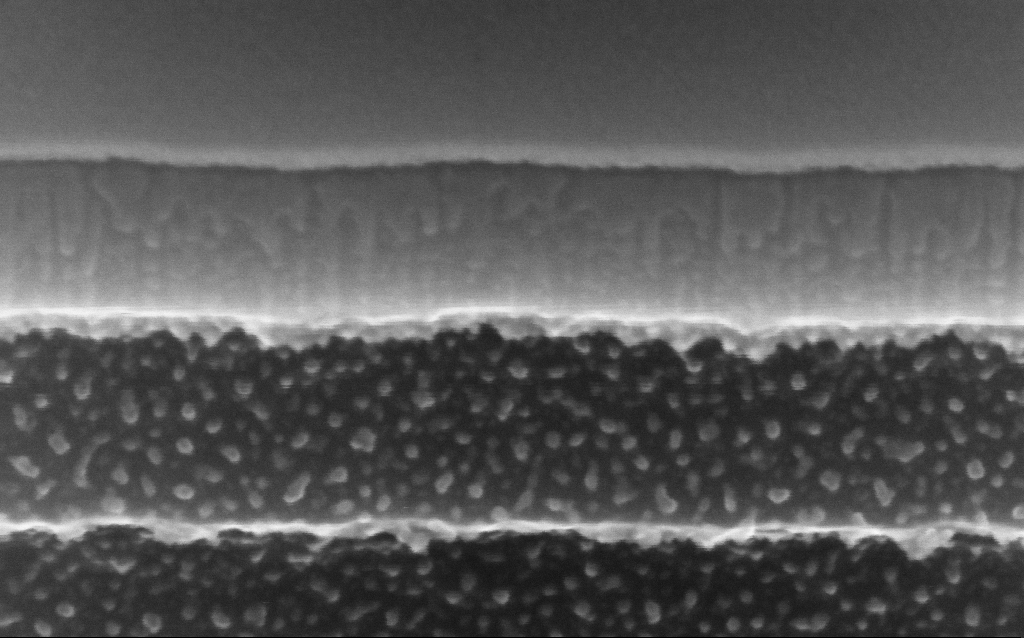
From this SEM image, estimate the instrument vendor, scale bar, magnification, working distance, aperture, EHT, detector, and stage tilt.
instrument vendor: Zeiss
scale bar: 100 nm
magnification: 423.35 K X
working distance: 4.8 mm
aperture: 30 µm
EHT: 10 kV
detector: InLens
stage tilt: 45°